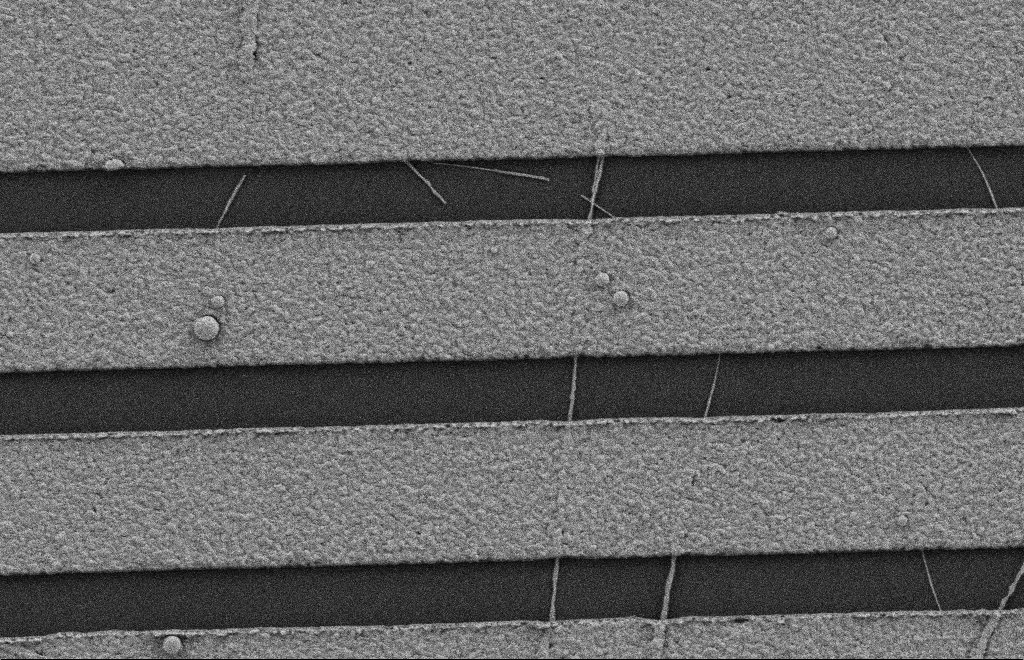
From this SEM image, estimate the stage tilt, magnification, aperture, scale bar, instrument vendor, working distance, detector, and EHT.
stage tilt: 0°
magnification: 18.43 K X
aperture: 20 µm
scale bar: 2000 nm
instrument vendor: Zeiss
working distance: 9 mm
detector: SE2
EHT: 2 kV